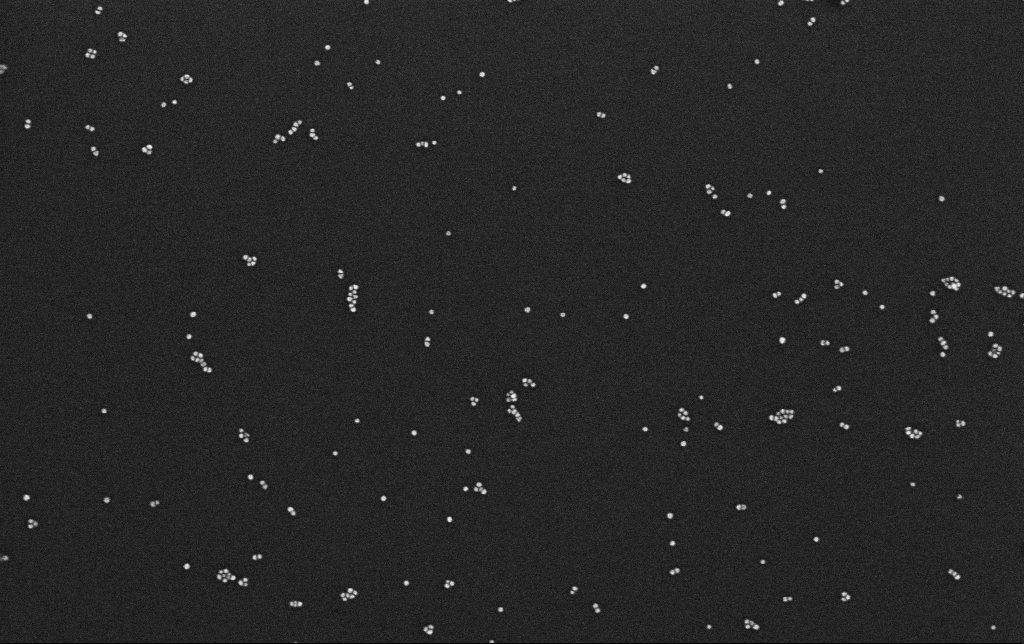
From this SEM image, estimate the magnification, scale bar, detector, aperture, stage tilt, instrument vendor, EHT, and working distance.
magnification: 120.18 K X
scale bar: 200 nm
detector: InLens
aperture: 30 µm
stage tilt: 0°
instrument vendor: Zeiss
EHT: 10 kV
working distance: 3.1 mm